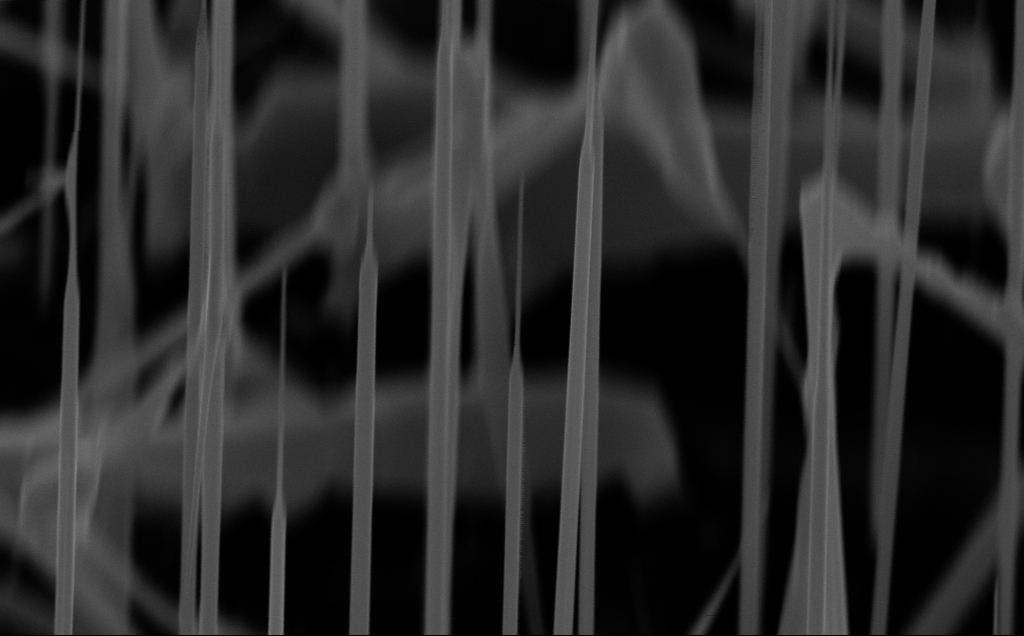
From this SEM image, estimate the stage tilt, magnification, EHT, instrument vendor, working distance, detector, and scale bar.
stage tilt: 45°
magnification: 71.68 K X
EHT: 10 kV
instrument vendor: Zeiss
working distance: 6 mm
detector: InLens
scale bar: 1000 nm